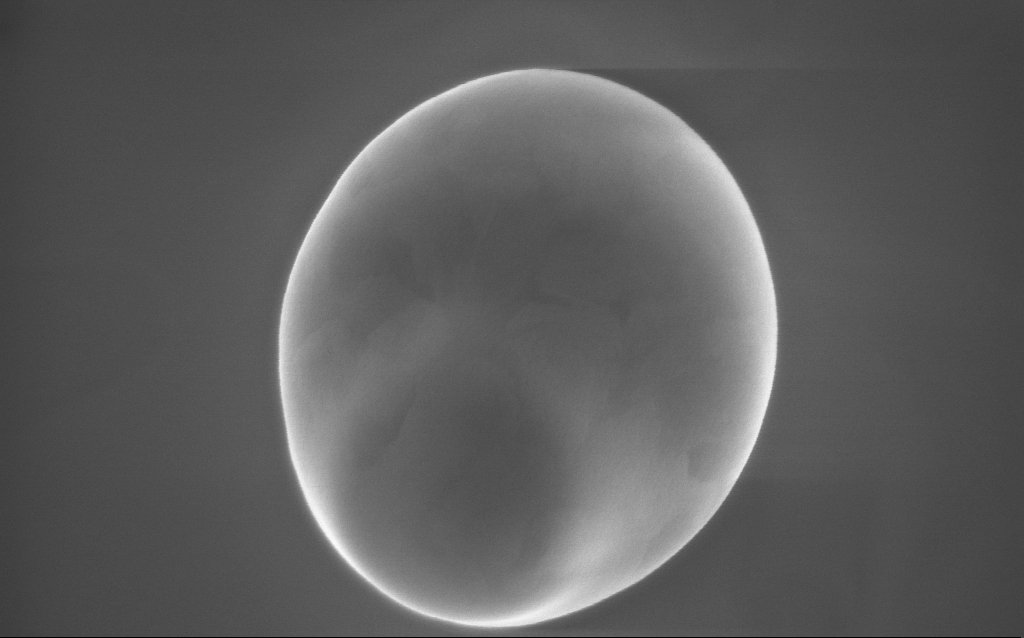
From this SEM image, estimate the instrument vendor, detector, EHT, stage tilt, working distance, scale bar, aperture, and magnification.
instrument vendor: Zeiss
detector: InLens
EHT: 10 kV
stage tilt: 0°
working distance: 2 mm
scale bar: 1000 nm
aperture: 30 µm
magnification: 71.1 K X